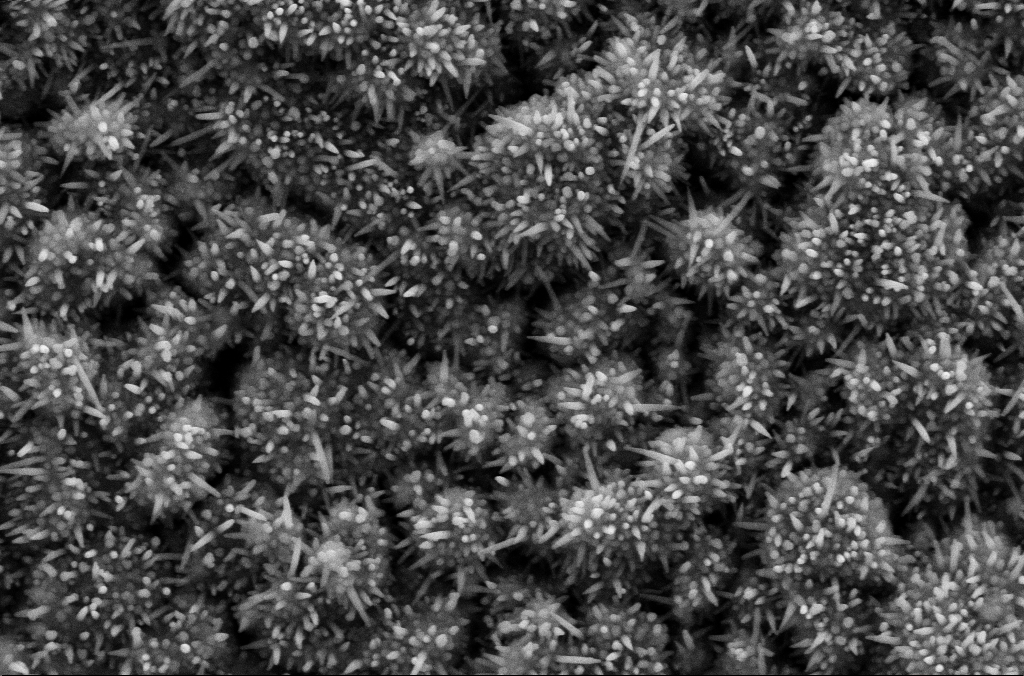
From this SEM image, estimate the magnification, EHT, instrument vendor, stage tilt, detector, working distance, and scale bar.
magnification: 45.76 K X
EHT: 10 kV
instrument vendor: Zeiss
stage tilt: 0.1°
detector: SE2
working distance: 5.2 mm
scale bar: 1000 nm